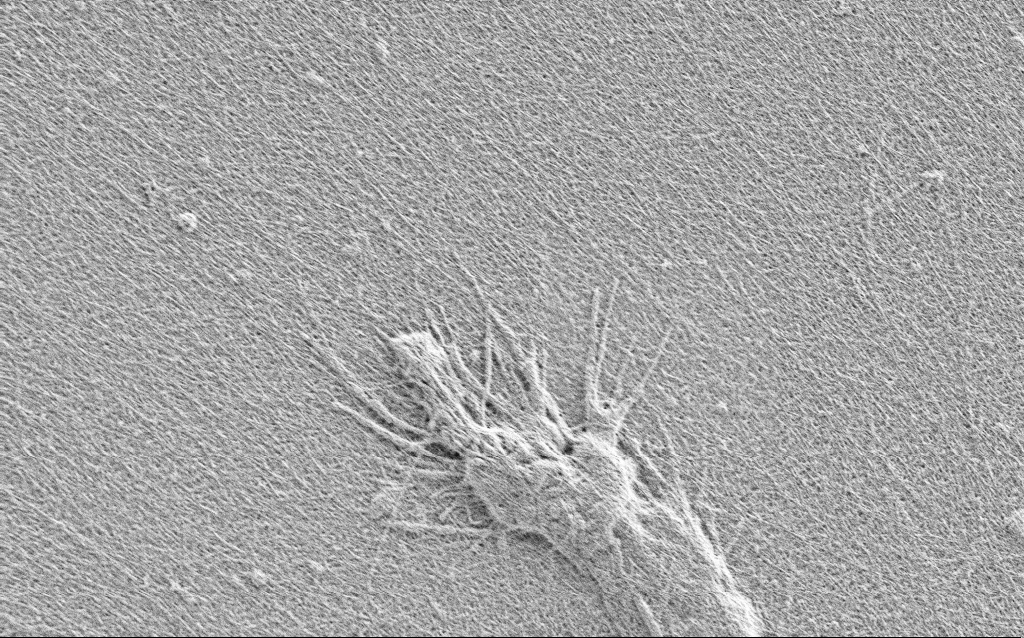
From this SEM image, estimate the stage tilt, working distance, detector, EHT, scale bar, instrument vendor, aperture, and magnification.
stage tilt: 0°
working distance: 3 mm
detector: SE2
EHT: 0.9 kV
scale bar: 2000 nm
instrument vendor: Zeiss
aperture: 30 µm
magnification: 10 K X